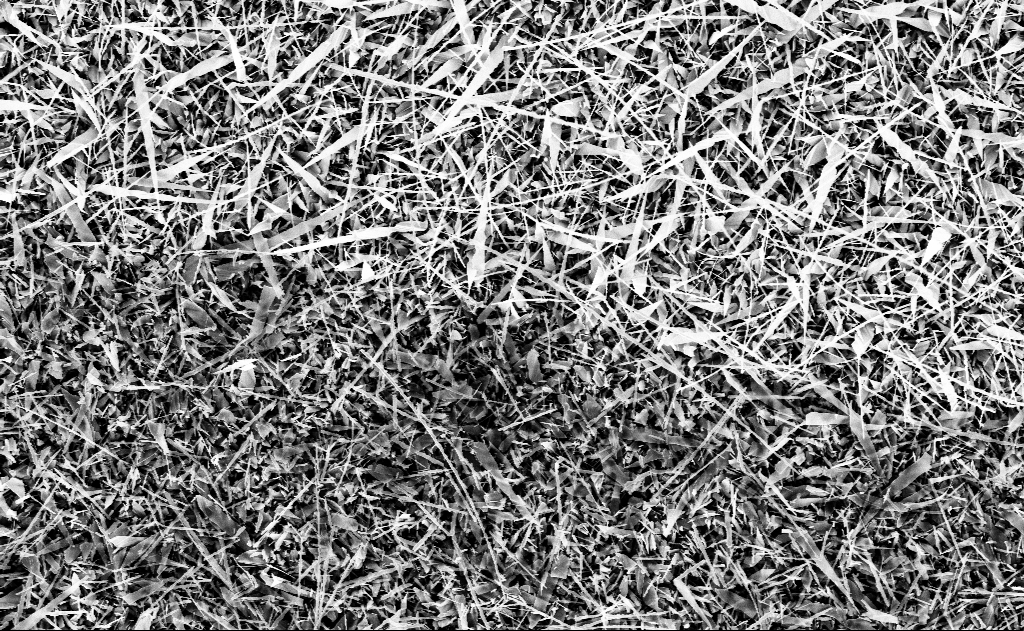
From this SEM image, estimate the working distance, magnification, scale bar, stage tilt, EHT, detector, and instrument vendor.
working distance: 13 mm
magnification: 5 K X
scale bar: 10000 nm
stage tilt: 0°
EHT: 10 kV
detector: InLens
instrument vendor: Zeiss